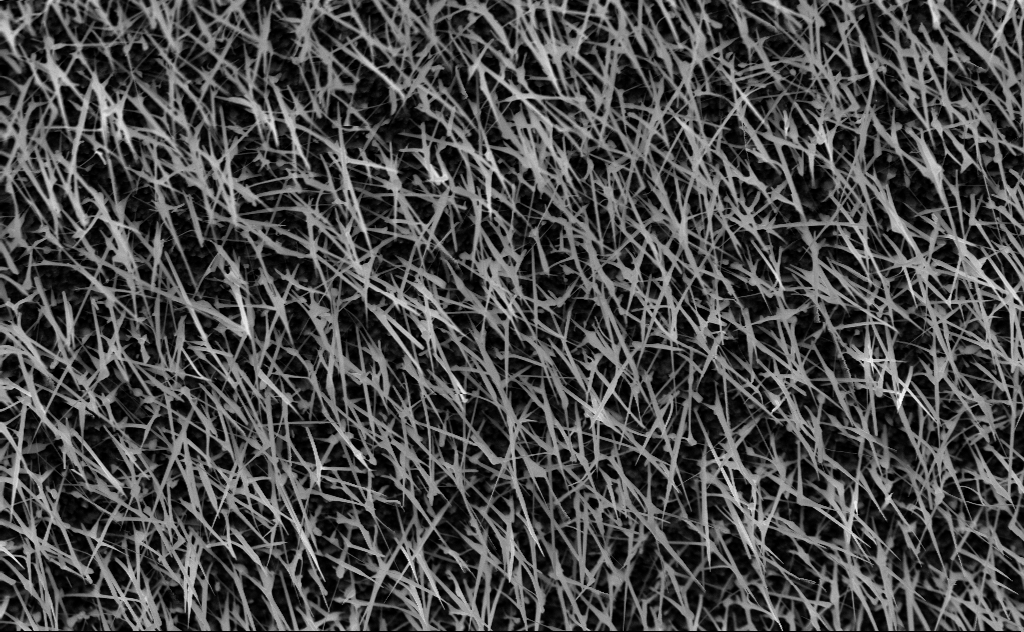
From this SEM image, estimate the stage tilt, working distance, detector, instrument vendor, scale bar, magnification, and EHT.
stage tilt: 45°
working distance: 5 mm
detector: InLens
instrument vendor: Zeiss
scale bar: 2000 nm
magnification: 20 K X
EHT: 10 kV